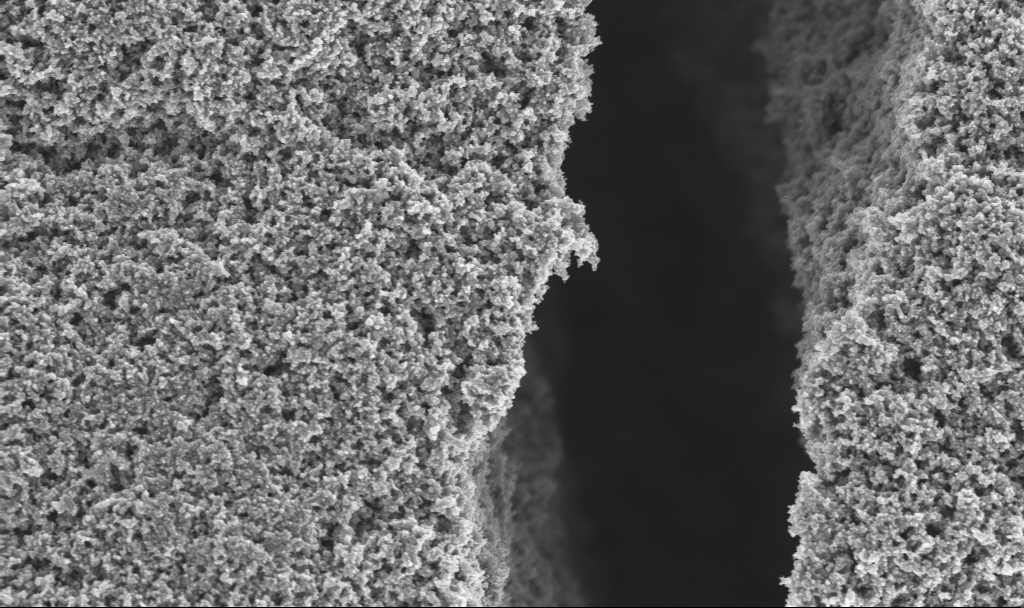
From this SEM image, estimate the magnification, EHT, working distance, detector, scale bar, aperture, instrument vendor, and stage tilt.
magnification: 32.58 K X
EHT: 3 kV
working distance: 2.4 mm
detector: InLens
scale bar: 1000 nm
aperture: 30 µm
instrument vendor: Zeiss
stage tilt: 0°